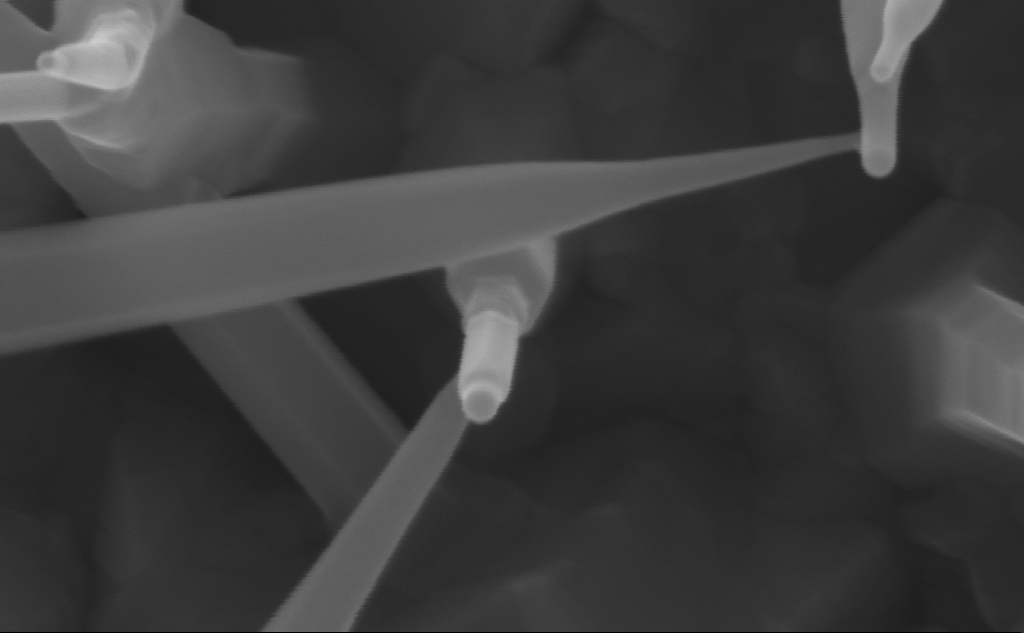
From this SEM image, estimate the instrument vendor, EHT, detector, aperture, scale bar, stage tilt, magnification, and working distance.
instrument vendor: Zeiss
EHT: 10 kV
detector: InLens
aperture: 30 µm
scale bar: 100 nm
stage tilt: -0°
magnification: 312.14 K X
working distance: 7 mm